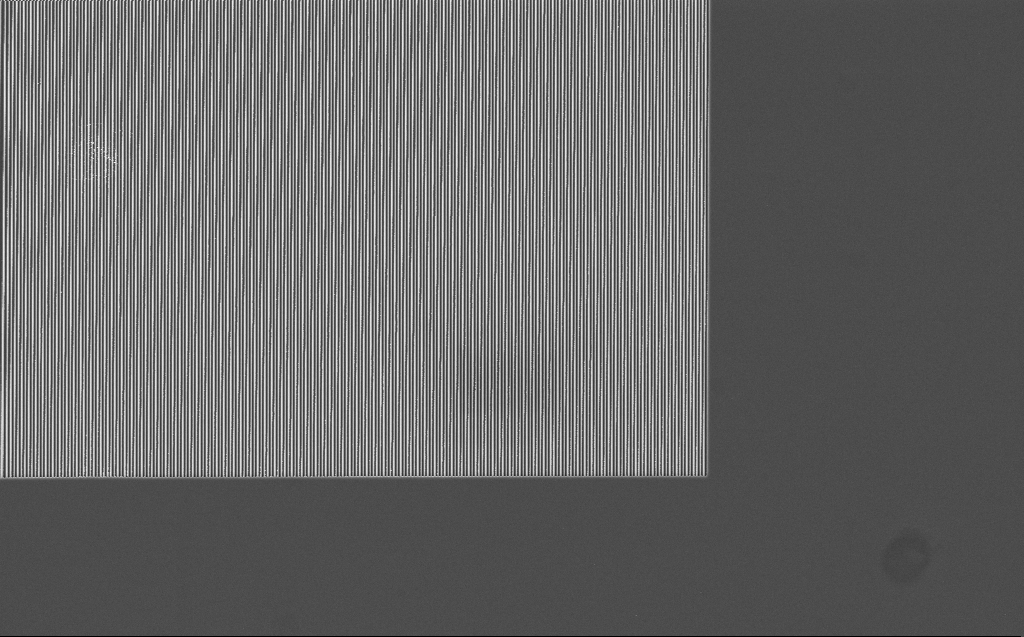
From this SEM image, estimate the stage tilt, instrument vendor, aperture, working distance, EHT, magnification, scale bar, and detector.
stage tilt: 0°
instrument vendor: Zeiss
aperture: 30 µm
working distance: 7 mm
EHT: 5 kV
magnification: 4.18 K X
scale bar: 10000 nm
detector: InLens